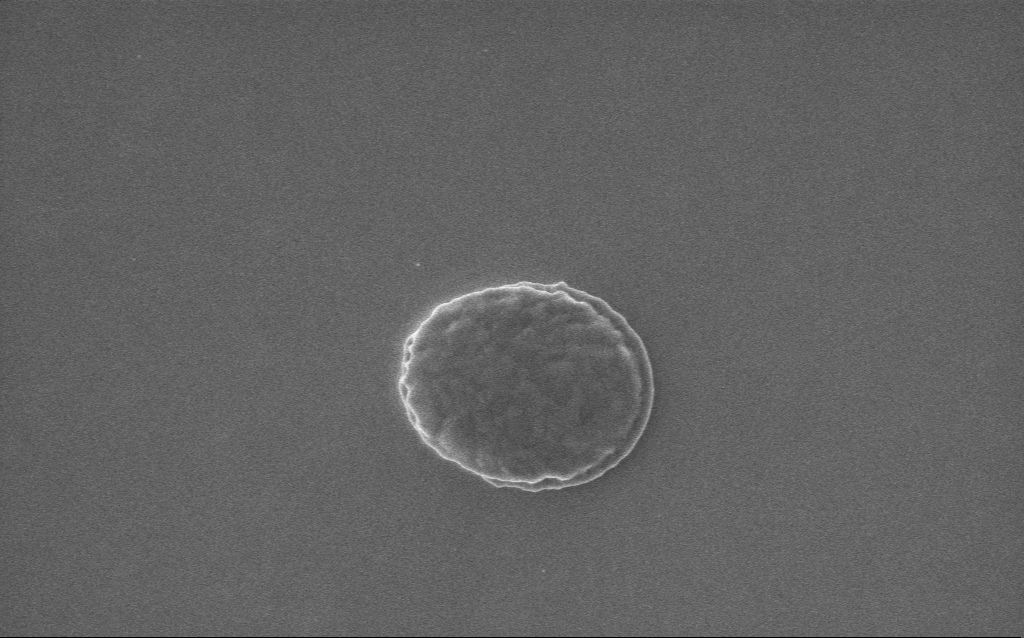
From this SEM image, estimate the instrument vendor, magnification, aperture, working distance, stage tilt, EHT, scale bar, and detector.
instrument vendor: Zeiss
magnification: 28.66 K X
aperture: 30 µm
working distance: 2 mm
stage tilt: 0°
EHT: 5 kV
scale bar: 2000 nm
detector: InLens